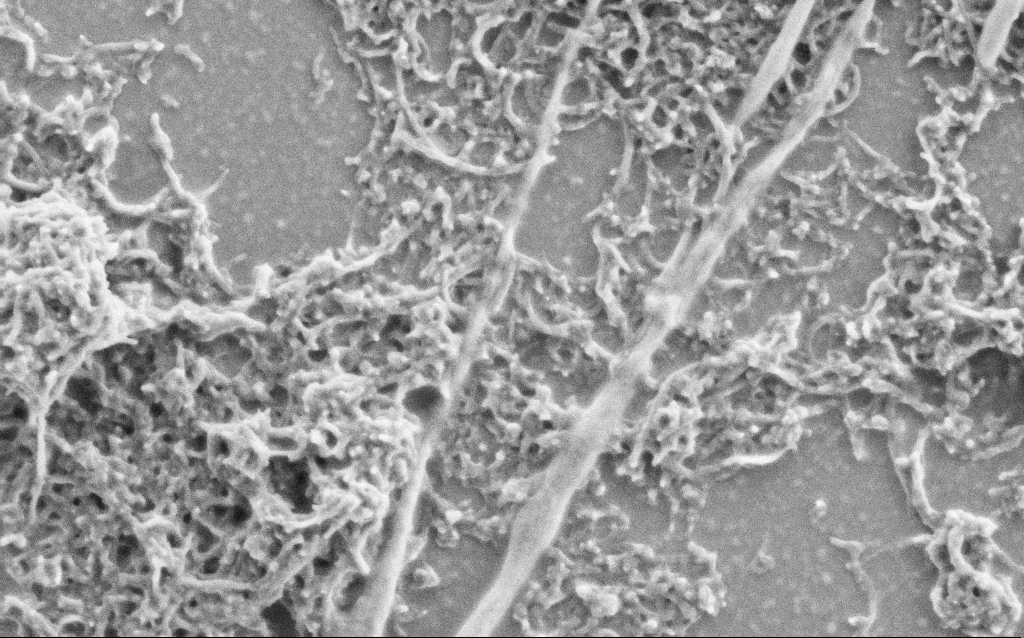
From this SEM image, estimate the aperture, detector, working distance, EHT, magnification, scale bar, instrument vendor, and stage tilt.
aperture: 30 µm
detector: SE2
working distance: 6.9 mm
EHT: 2 kV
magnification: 50 K X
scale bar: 1000 nm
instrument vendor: Zeiss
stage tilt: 0°